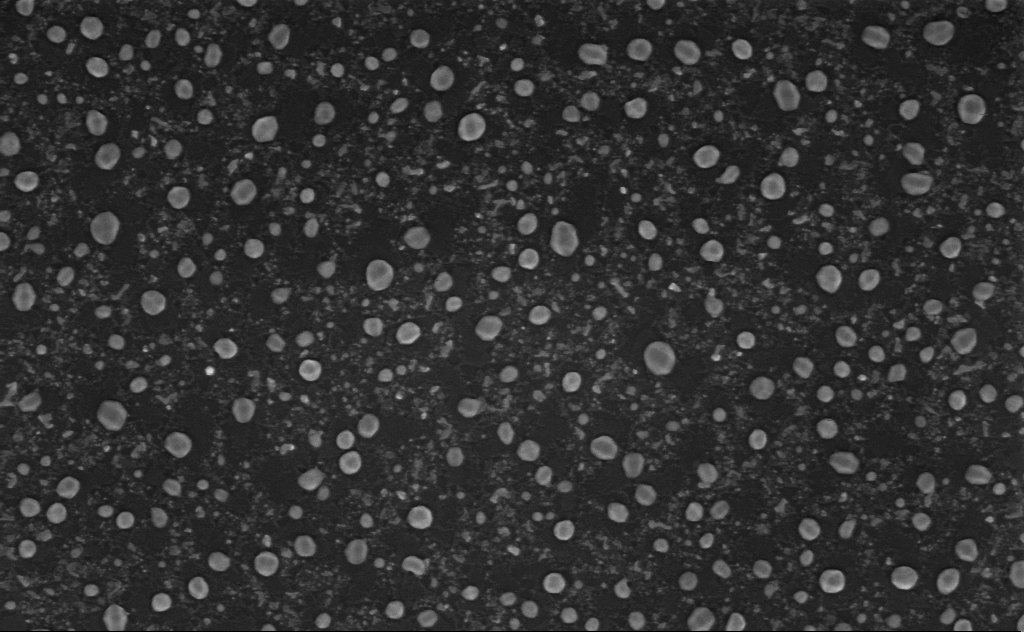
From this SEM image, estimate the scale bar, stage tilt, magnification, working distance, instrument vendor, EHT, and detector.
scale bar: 200 nm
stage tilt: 0°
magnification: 80 K X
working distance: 4 mm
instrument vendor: Zeiss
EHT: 3 kV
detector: InLens